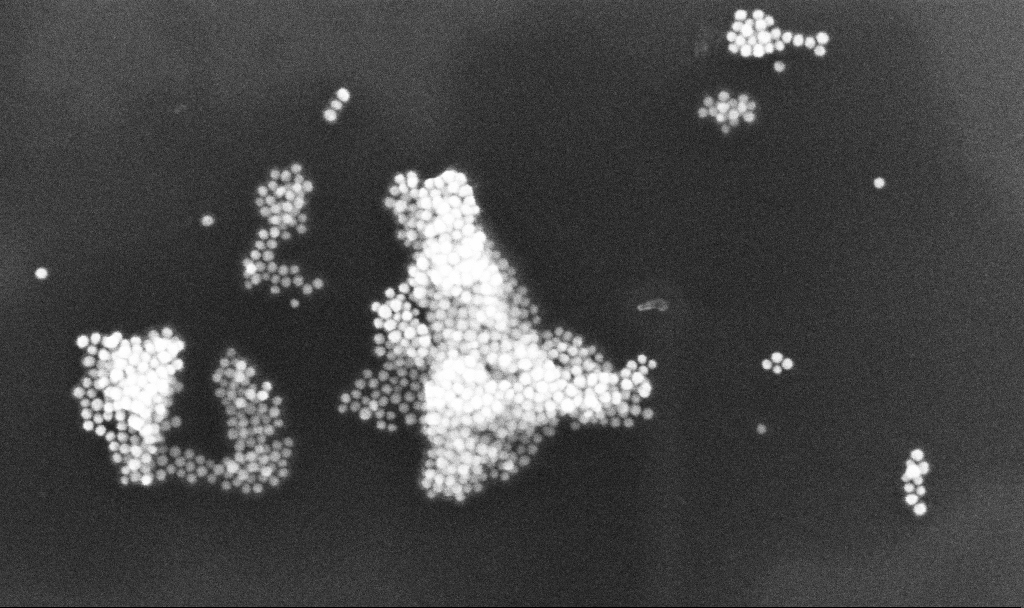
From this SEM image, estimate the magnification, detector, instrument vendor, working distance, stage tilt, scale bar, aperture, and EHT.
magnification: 200 K X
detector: InLens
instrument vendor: Zeiss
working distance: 3.3 mm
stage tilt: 0°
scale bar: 200 nm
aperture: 30 µm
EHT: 10 kV